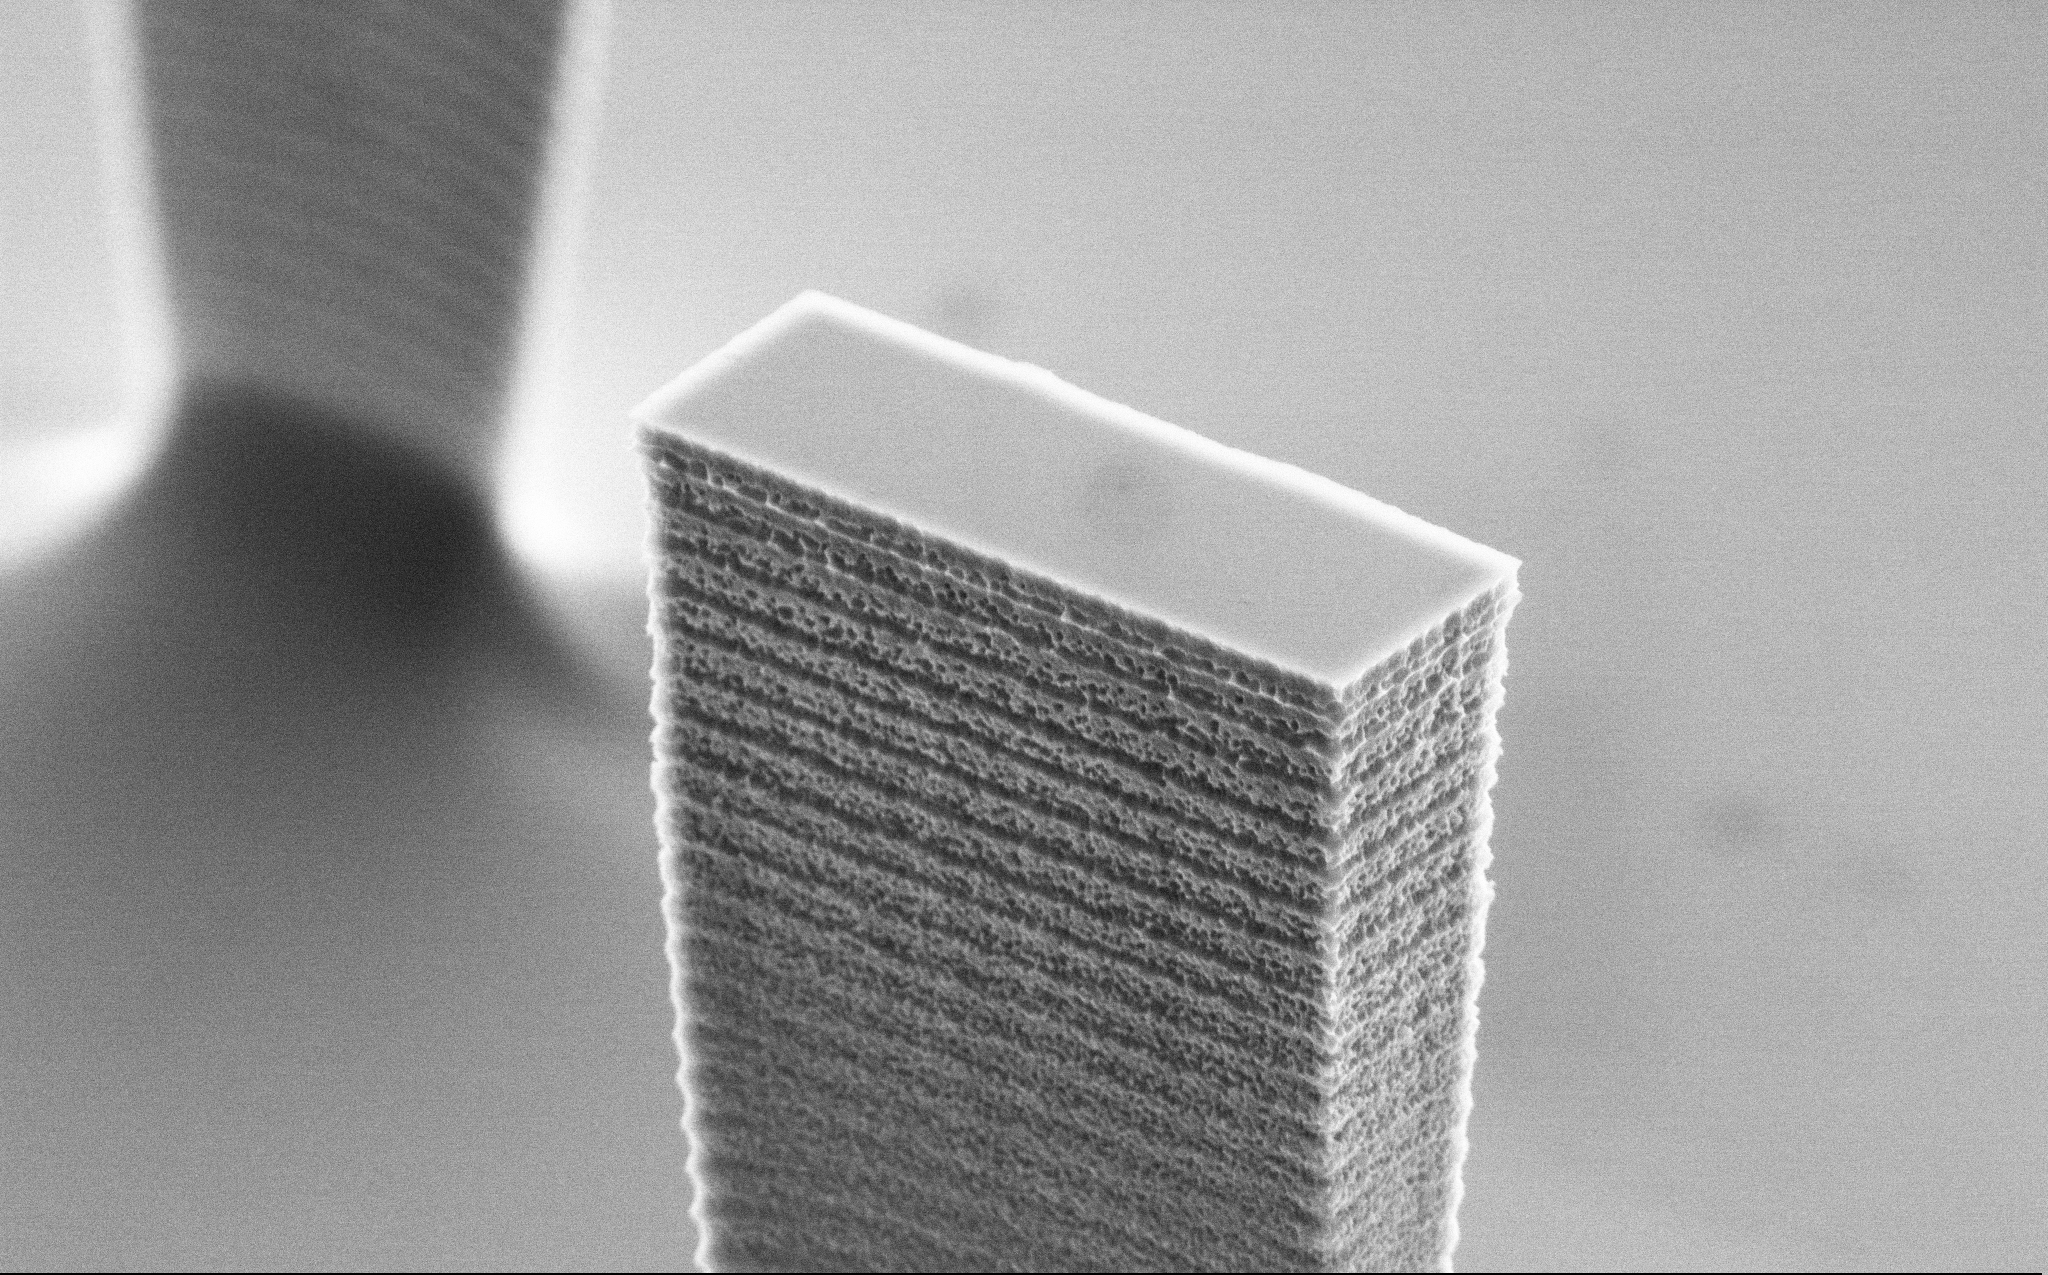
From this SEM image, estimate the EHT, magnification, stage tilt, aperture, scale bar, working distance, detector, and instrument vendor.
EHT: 5 kV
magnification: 21.29 K X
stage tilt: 60°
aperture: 30 µm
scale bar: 1000 nm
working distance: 9.7 mm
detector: SE2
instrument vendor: Zeiss